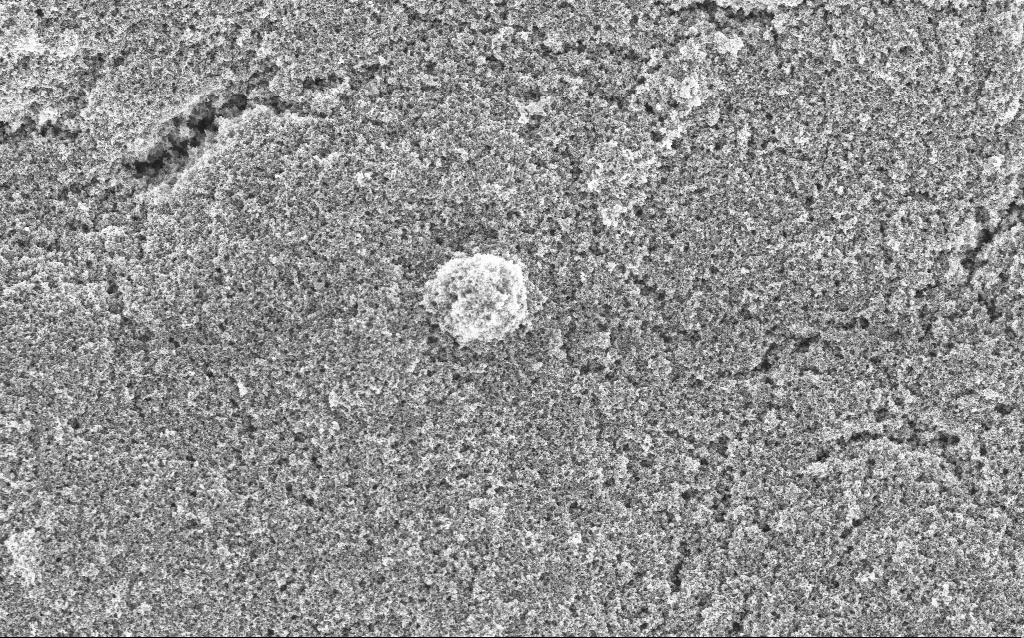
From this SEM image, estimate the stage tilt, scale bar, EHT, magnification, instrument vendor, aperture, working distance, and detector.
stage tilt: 0°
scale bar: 1000 nm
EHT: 5 kV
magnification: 15.33 K X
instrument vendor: Zeiss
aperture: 30 µm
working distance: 4.4 mm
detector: InLens